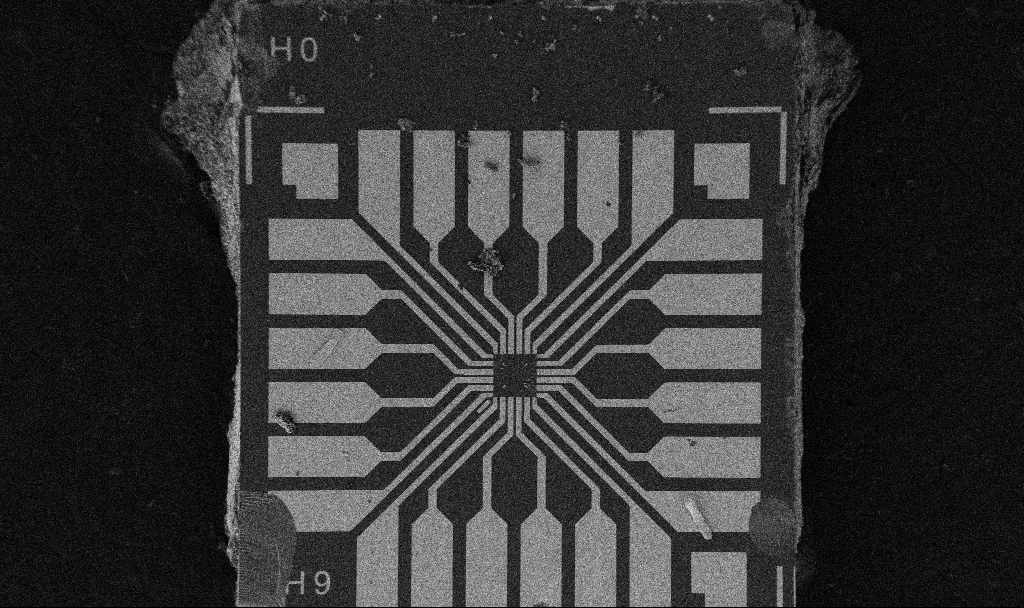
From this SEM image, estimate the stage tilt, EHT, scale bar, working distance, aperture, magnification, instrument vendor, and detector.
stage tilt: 0°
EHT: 5 kV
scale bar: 200000 nm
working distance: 10.7 mm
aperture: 30 µm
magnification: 0.1 K X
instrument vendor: Zeiss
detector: SE2